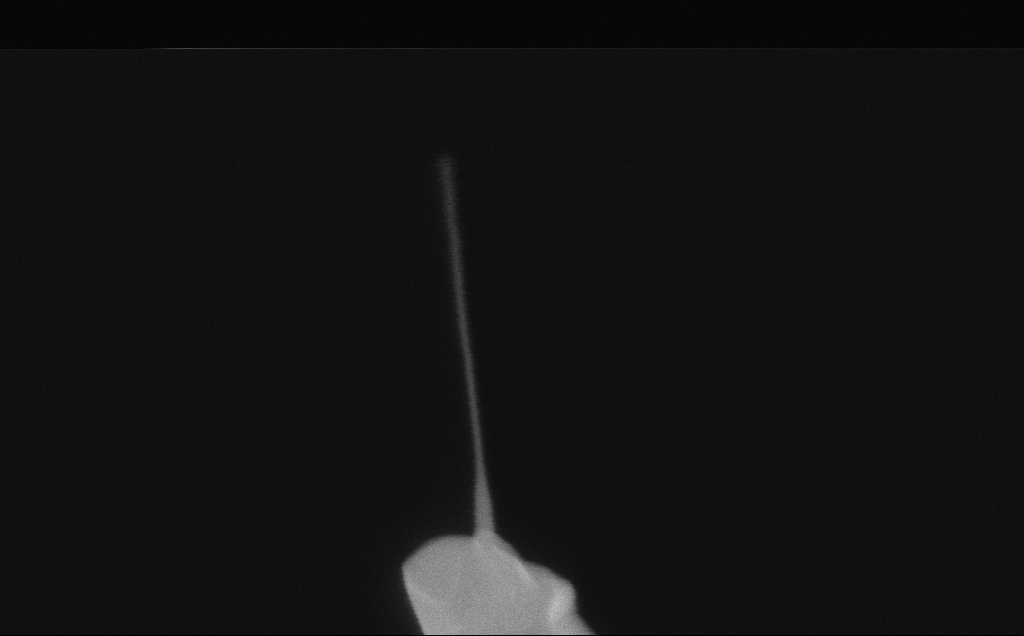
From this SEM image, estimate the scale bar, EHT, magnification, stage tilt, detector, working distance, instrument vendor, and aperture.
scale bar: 200 nm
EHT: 10 kV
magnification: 257.27 K X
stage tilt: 0°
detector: InLens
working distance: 6 mm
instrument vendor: Zeiss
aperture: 30 µm